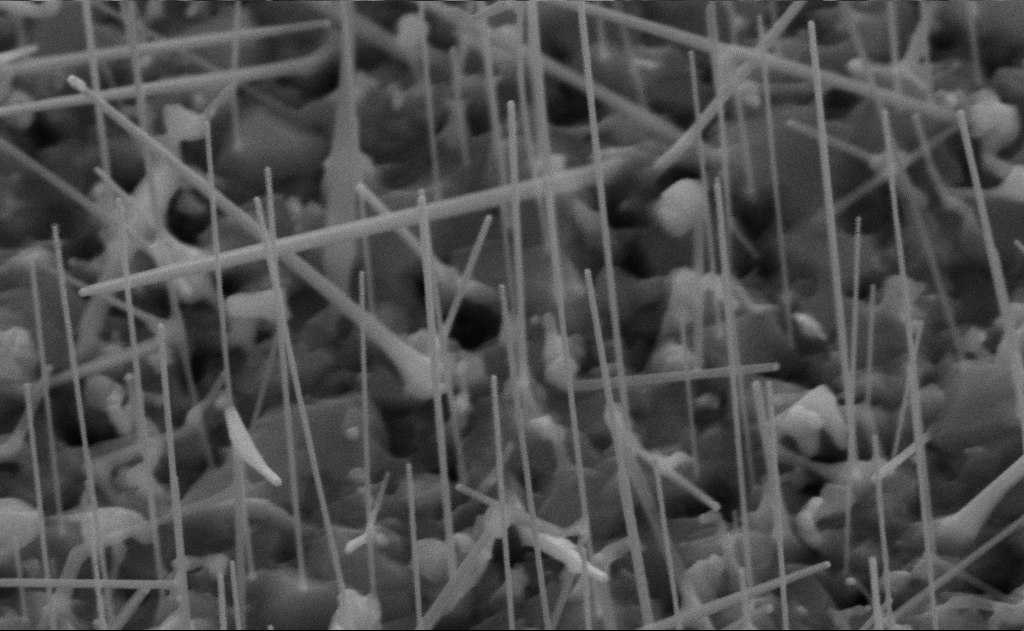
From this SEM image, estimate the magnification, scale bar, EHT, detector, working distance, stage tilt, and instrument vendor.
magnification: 100 K X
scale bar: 200 nm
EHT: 10 kV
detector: SE2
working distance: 11 mm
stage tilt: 45°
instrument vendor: Zeiss